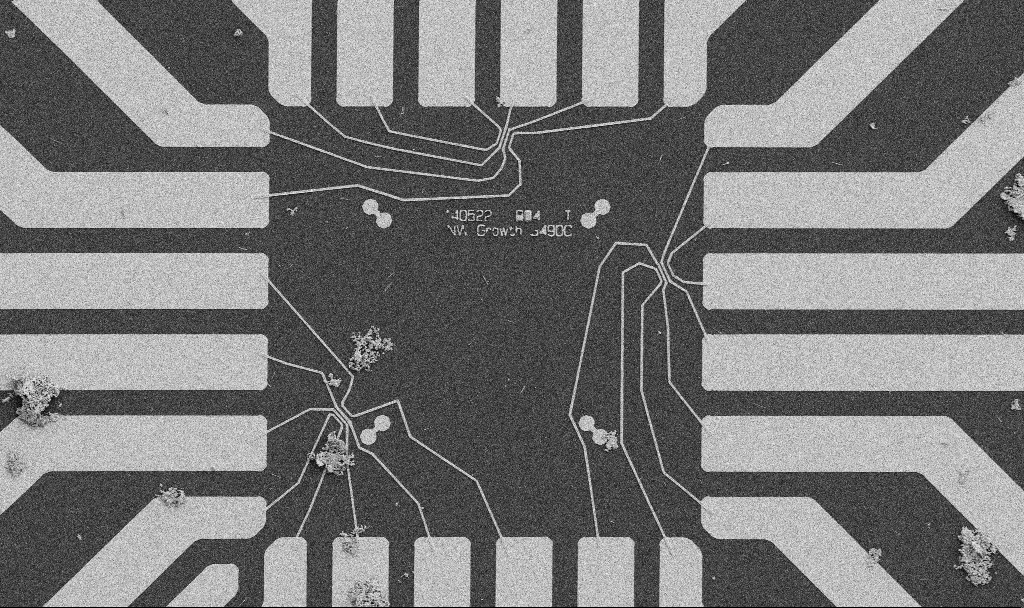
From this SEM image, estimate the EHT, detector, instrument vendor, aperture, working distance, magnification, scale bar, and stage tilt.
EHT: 5 kV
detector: SE2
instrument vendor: Zeiss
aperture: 30 µm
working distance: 10.7 mm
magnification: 1 K X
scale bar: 20000 nm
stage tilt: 0°